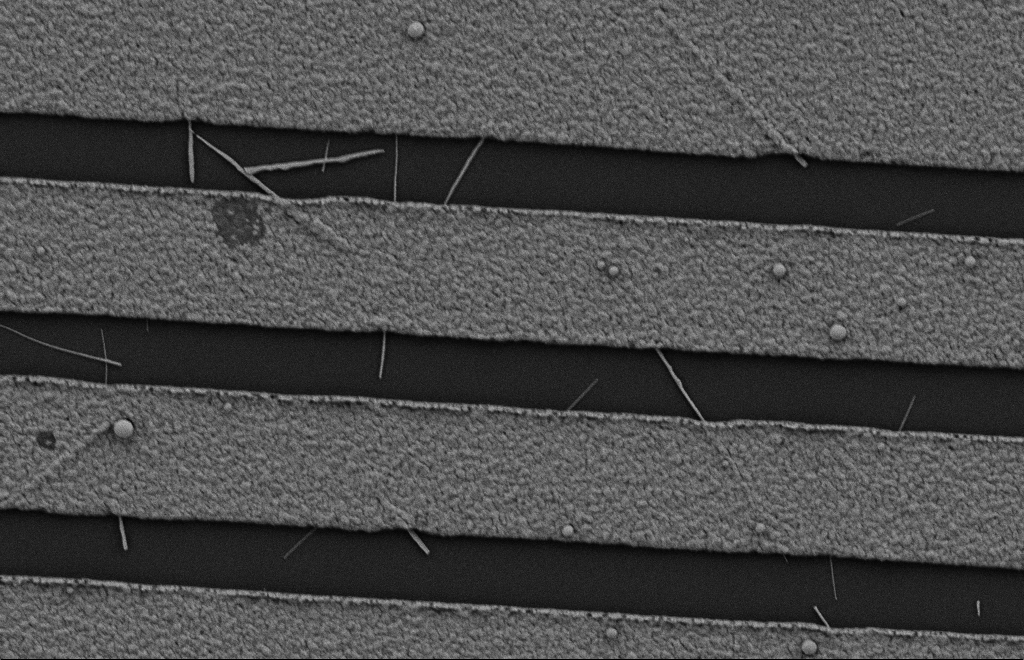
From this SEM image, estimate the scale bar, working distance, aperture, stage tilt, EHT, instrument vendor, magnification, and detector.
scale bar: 2000 nm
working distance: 10 mm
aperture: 20 µm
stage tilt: -0.3°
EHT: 2 kV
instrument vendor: Zeiss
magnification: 18.28 K X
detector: SE2